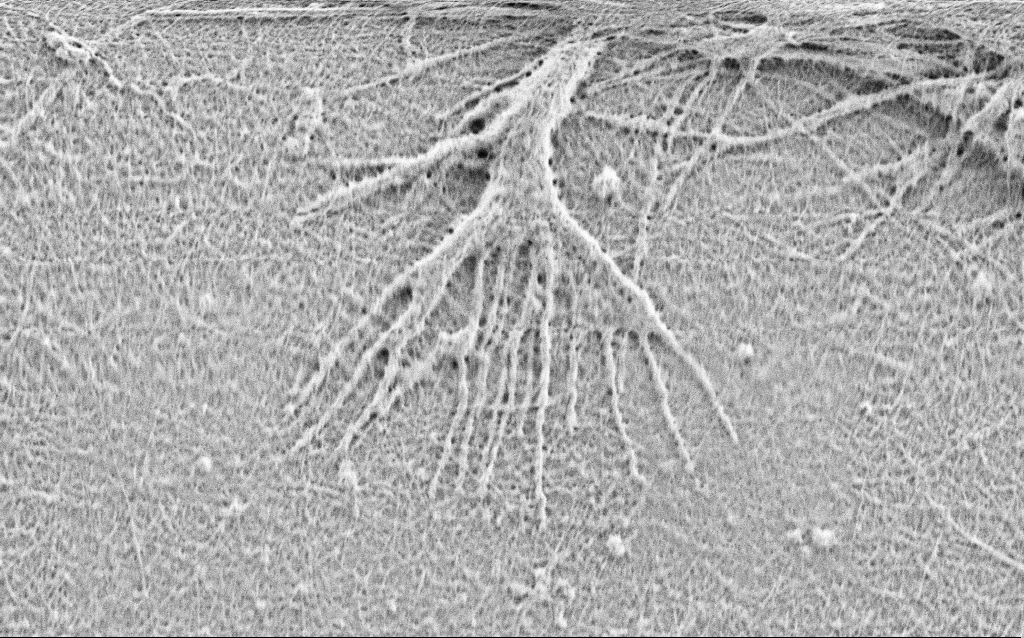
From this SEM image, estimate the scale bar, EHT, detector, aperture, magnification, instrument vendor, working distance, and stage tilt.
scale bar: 1000 nm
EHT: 0.9 kV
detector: SE2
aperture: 30 µm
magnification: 20 K X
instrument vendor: Zeiss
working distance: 3 mm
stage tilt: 0°